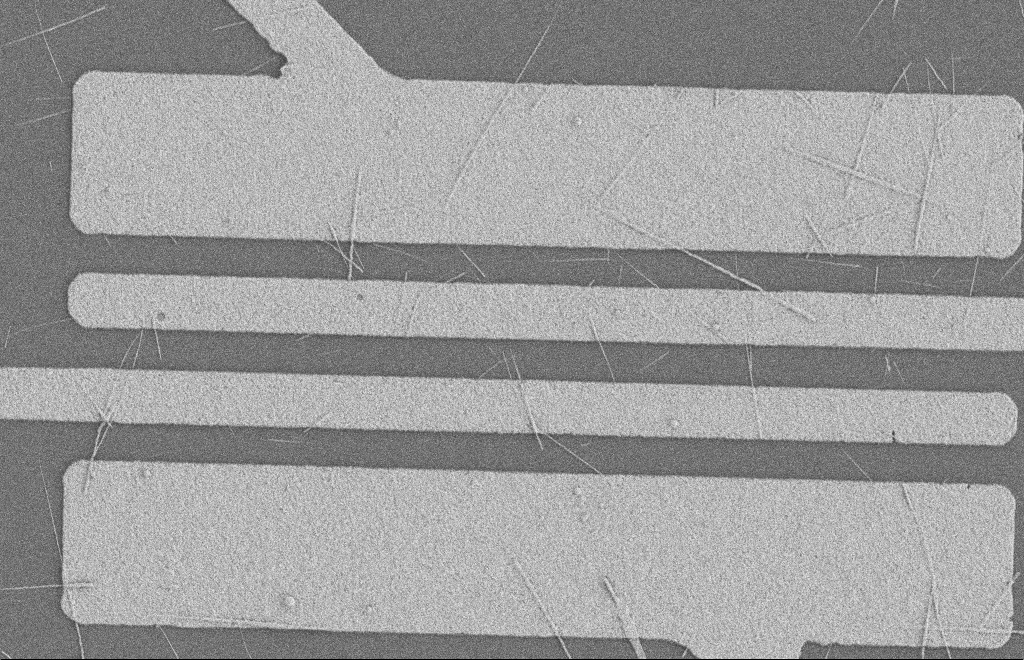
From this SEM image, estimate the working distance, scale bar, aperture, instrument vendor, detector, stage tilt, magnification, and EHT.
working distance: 8 mm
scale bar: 2000 nm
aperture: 20 µm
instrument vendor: Zeiss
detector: SE2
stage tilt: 0°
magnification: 5.73 K X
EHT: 2 kV